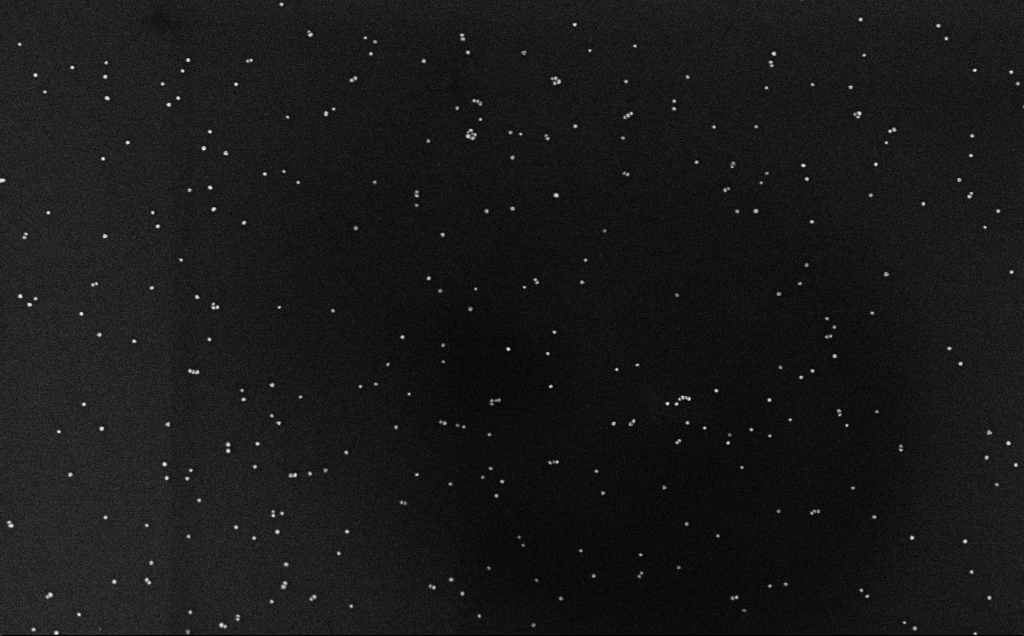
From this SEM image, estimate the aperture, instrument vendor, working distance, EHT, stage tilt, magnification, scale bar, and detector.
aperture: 30 µm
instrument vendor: Zeiss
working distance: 3.2 mm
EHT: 10 kV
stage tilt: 0°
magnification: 100 K X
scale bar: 200 nm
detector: InLens